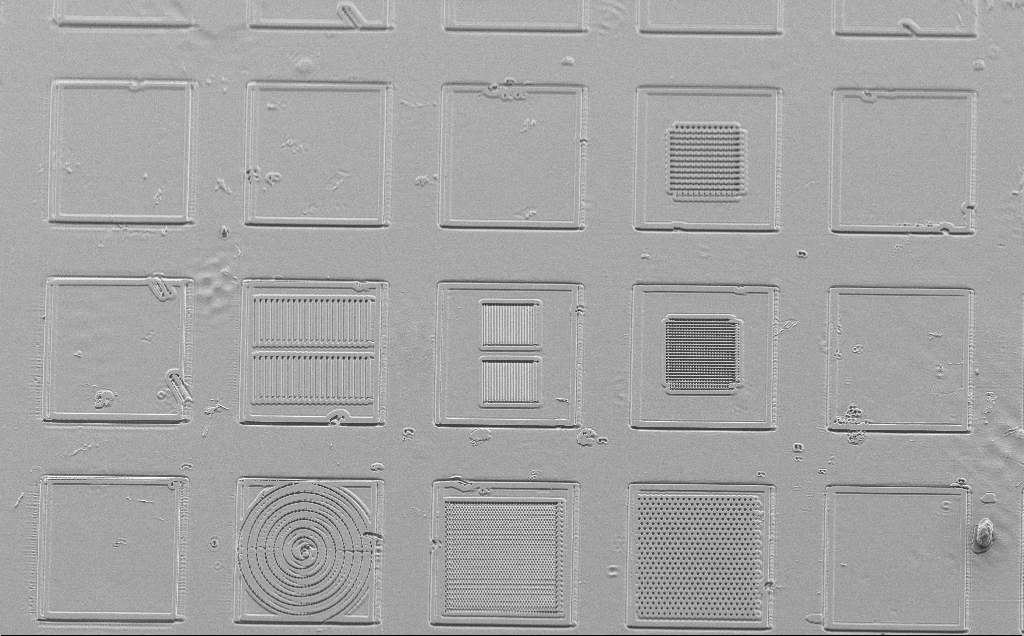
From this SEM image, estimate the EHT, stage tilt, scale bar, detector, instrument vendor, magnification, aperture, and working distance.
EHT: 5 kV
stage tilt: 45°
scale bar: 100000 nm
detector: SE2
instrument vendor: Zeiss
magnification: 0.506 K X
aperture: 30 µm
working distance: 10 mm